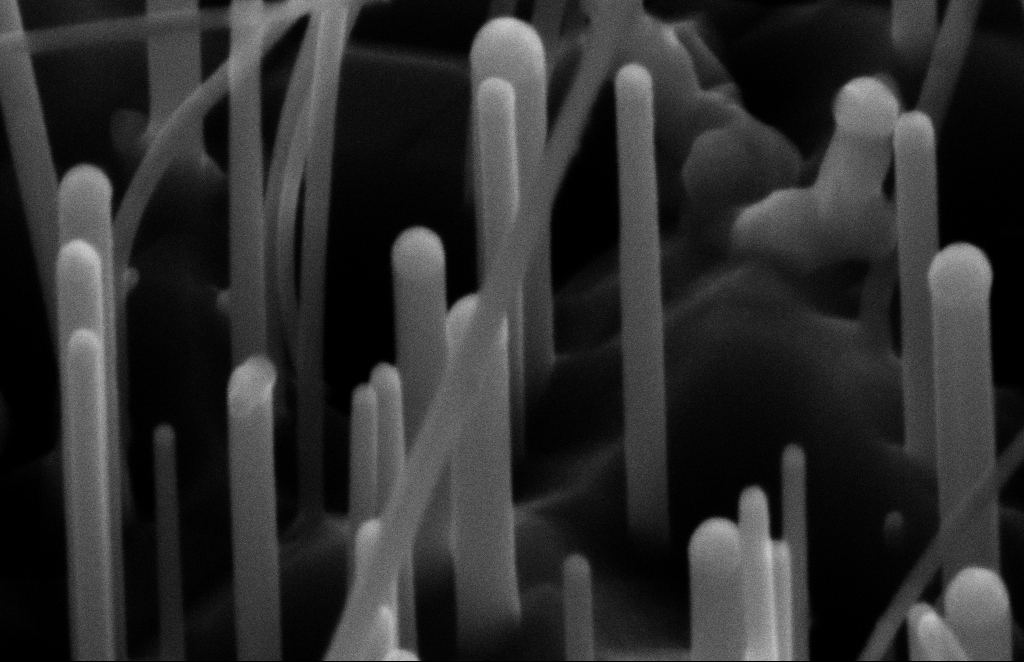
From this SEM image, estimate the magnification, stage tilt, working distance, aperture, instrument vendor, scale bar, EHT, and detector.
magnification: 300 K X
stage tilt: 45°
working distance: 15 mm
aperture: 20 µm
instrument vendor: Zeiss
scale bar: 100 nm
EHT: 10 kV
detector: SE2